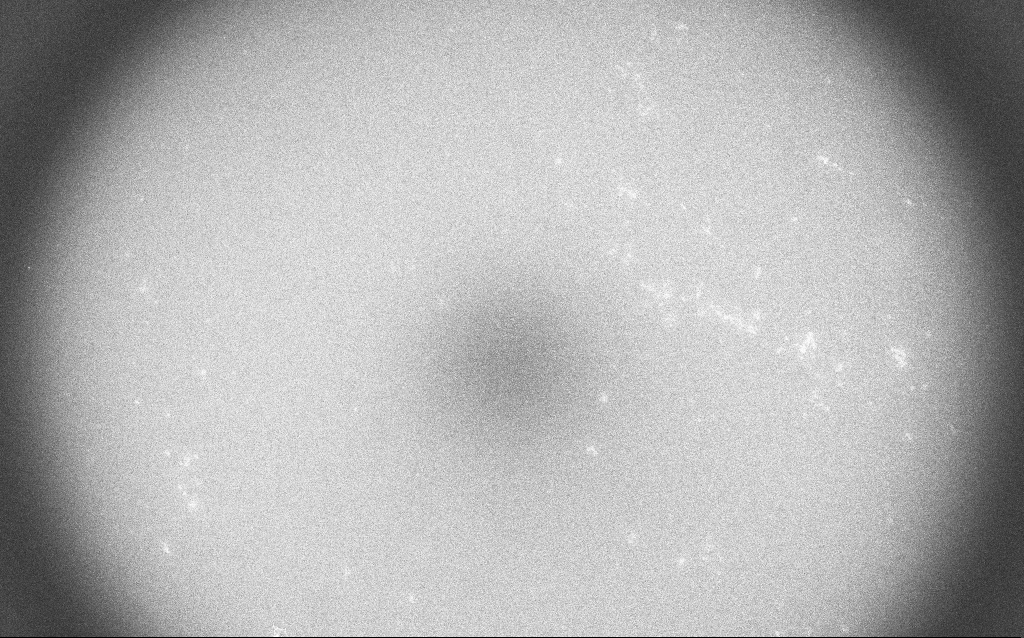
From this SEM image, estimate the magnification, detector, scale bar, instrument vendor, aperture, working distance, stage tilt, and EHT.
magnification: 0.2 K X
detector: InLens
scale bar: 200000 nm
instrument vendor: Zeiss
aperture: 30 µm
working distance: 3.3 mm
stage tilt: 0°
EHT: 20 kV